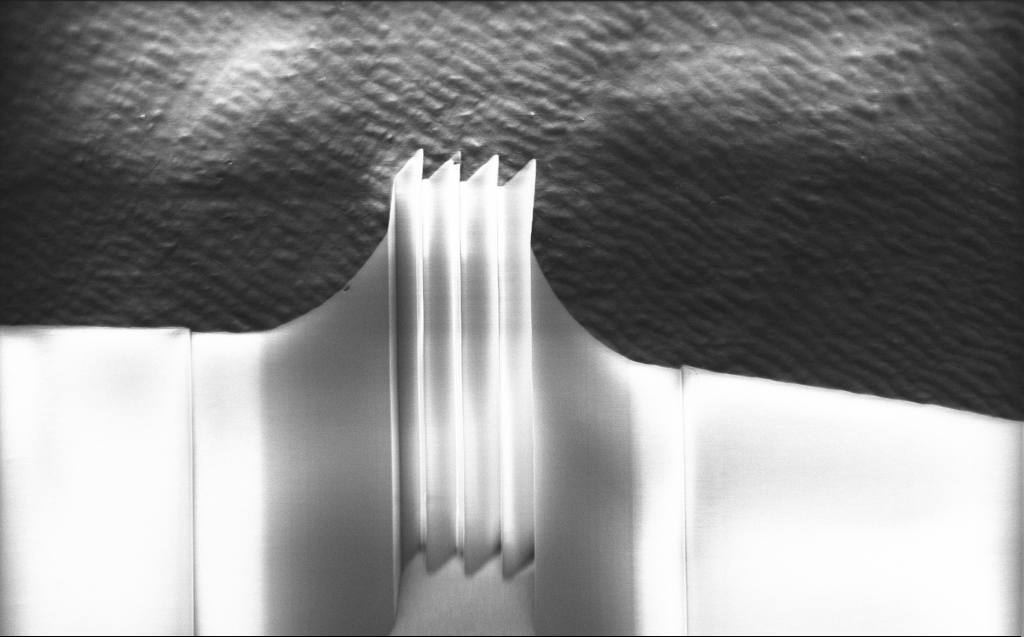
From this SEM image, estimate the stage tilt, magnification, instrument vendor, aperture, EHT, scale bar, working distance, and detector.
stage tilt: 44.9°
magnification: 1.21 K X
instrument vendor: Zeiss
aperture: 30 µm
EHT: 1 kV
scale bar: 10000 nm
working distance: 7 mm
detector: InLens